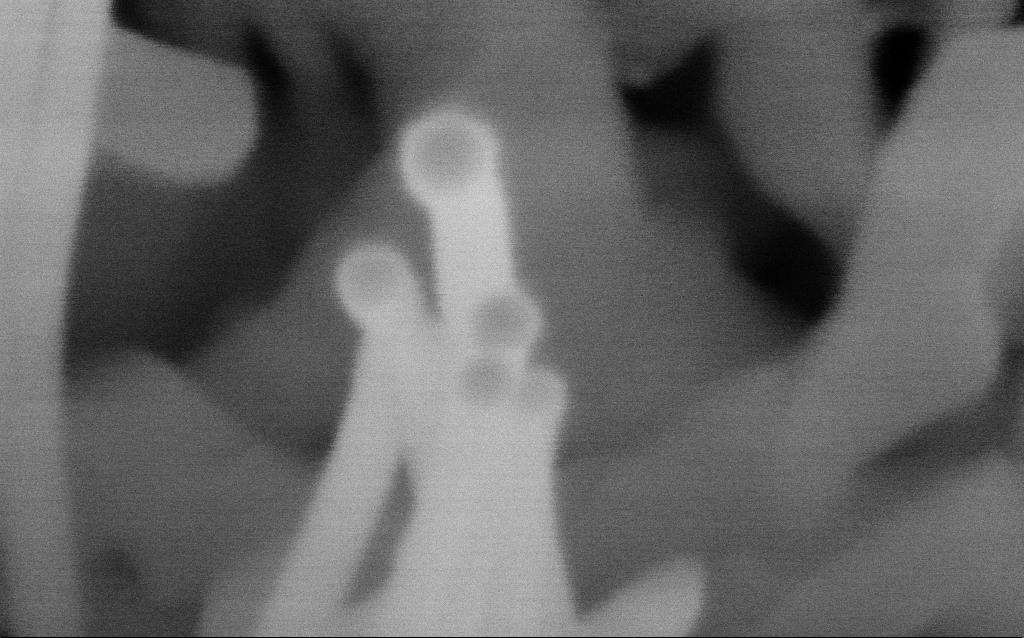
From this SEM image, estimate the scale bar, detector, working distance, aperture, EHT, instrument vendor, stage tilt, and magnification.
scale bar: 20 nm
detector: InLens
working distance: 7.3 mm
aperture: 30 µm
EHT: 10 kV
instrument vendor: Zeiss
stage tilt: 35°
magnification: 973.39 K X